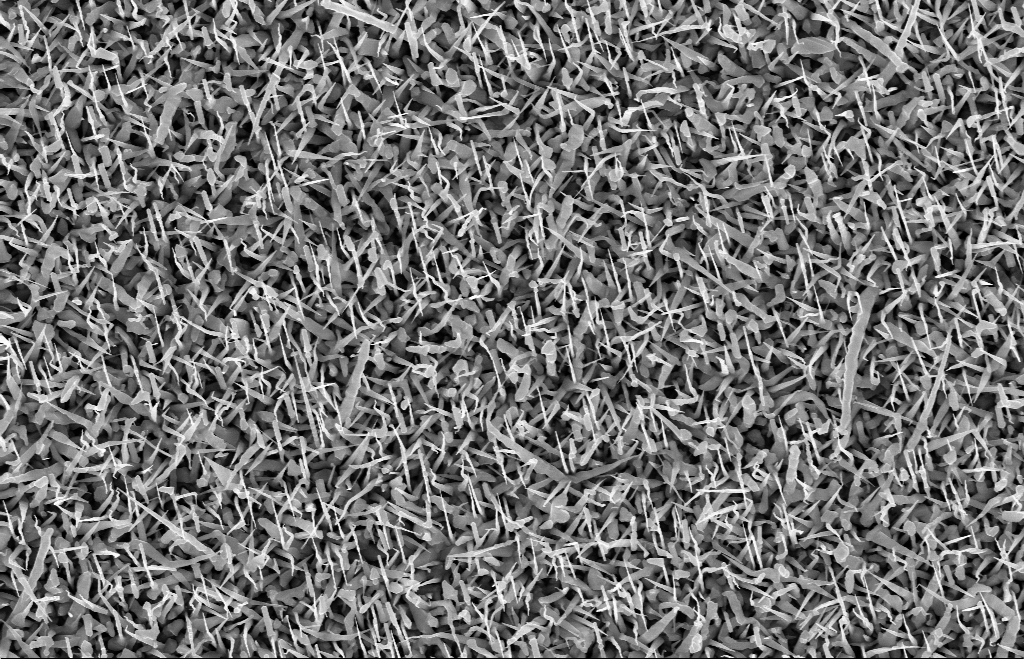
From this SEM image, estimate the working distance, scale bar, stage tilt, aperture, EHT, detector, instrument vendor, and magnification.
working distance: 8 mm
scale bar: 2000 nm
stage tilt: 0°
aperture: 30 µm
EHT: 10 kV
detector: InLens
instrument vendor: Zeiss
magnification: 20 K X